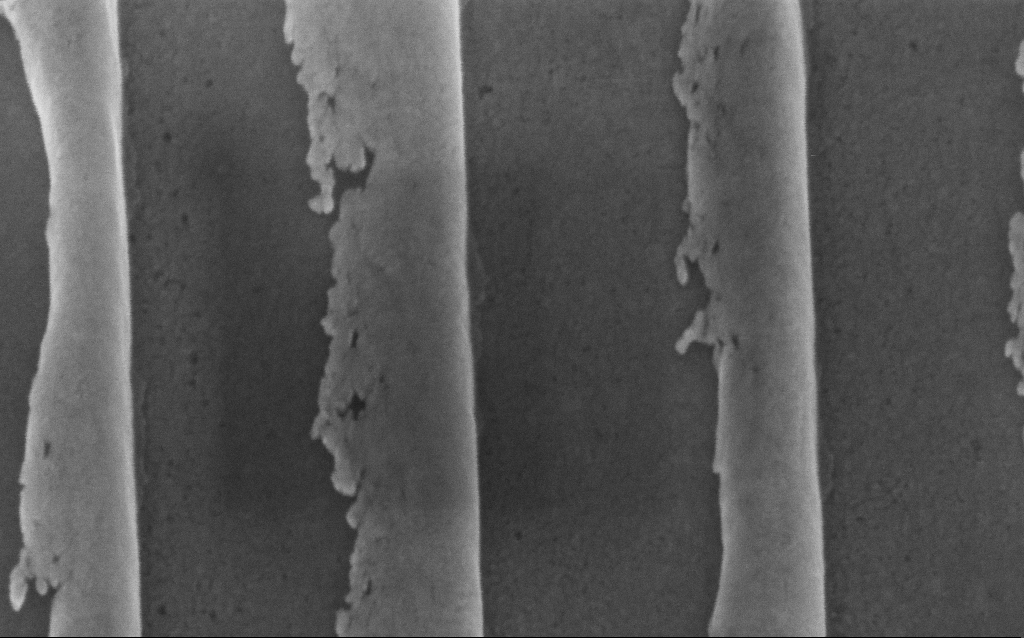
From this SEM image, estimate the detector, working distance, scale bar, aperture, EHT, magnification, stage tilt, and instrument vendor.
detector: InLens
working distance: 7 mm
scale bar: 200 nm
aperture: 30 µm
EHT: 10 kV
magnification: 253.54 K X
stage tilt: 45°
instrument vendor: Zeiss